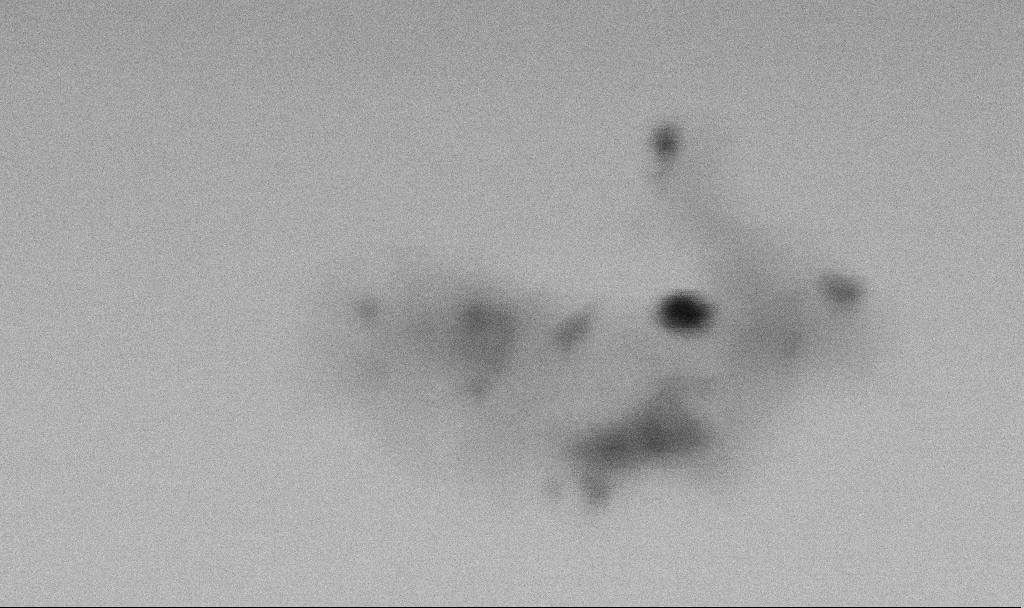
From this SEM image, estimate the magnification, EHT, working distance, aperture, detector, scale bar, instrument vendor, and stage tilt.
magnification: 324.9 K X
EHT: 2 kV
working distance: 6.5 mm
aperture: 30 µm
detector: SE2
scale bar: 100 nm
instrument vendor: Zeiss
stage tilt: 0°